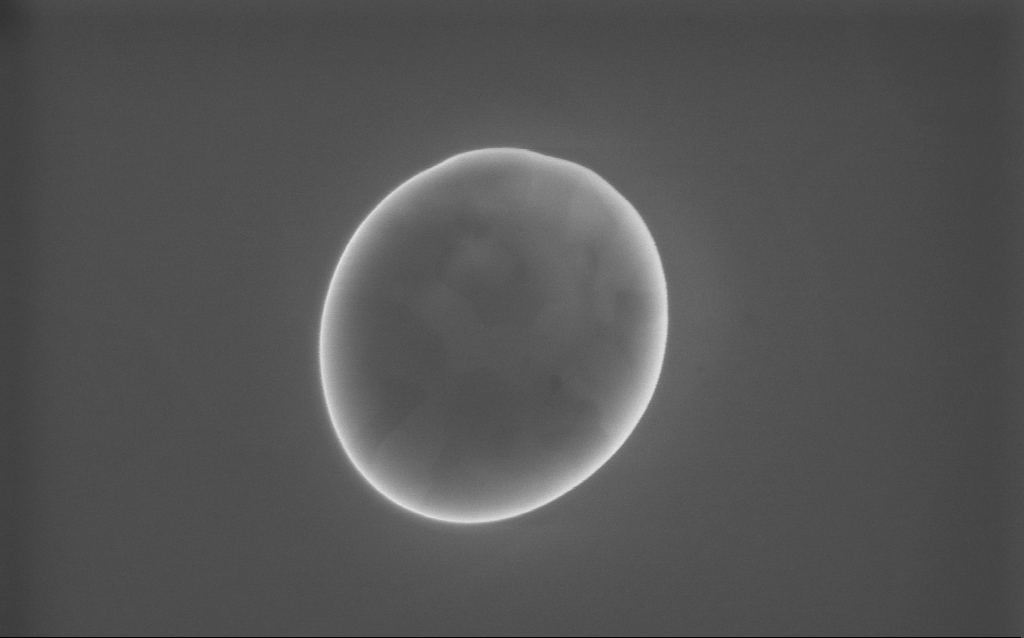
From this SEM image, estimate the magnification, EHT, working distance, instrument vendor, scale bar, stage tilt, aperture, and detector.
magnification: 69.88 K X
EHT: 10 kV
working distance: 2 mm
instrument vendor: Zeiss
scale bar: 1000 nm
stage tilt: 0°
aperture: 30 µm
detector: InLens